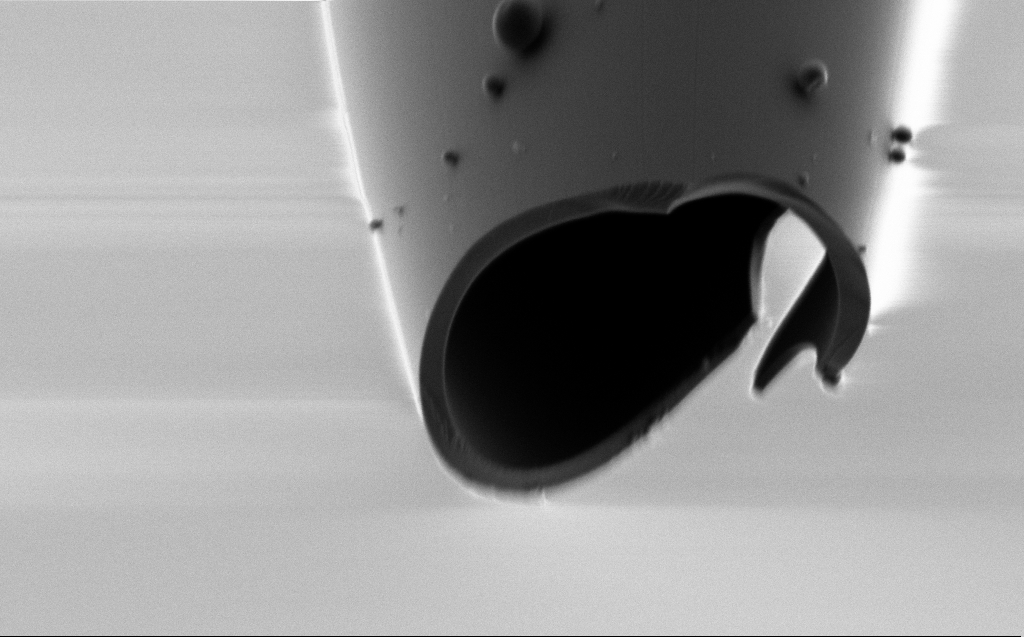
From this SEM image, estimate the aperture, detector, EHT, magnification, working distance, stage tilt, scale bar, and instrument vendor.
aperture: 30 µm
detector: SE2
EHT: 1 kV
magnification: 30.61 K X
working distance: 5 mm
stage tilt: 45.1°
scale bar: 2000 nm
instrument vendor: Zeiss